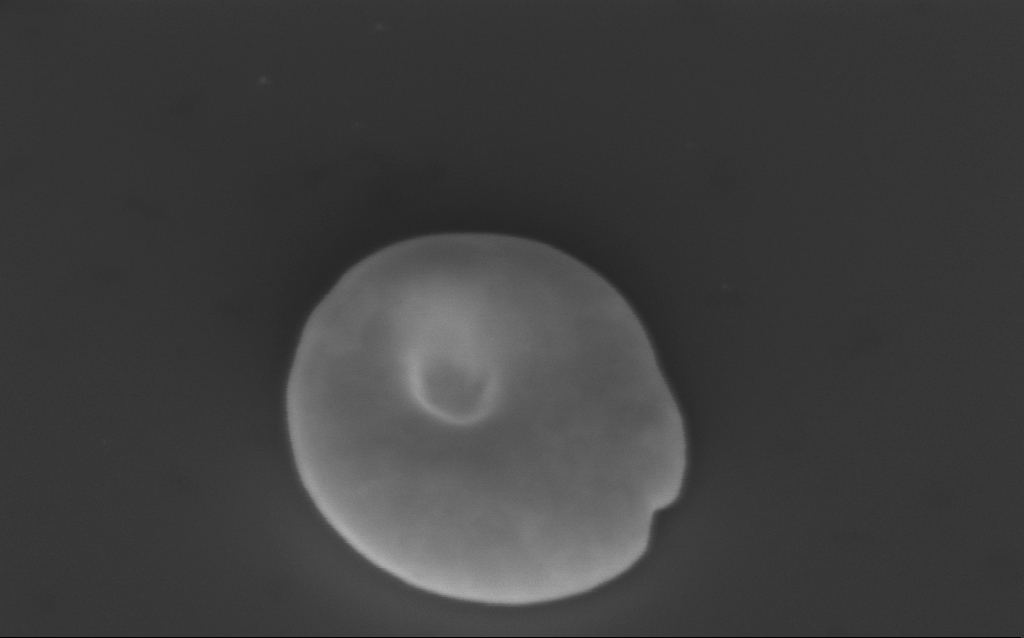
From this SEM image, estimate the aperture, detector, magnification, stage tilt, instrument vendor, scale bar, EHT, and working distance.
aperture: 30 µm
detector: InLens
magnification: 127.72 K X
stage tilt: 40°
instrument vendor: Zeiss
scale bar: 200 nm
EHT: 3 kV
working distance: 5 mm